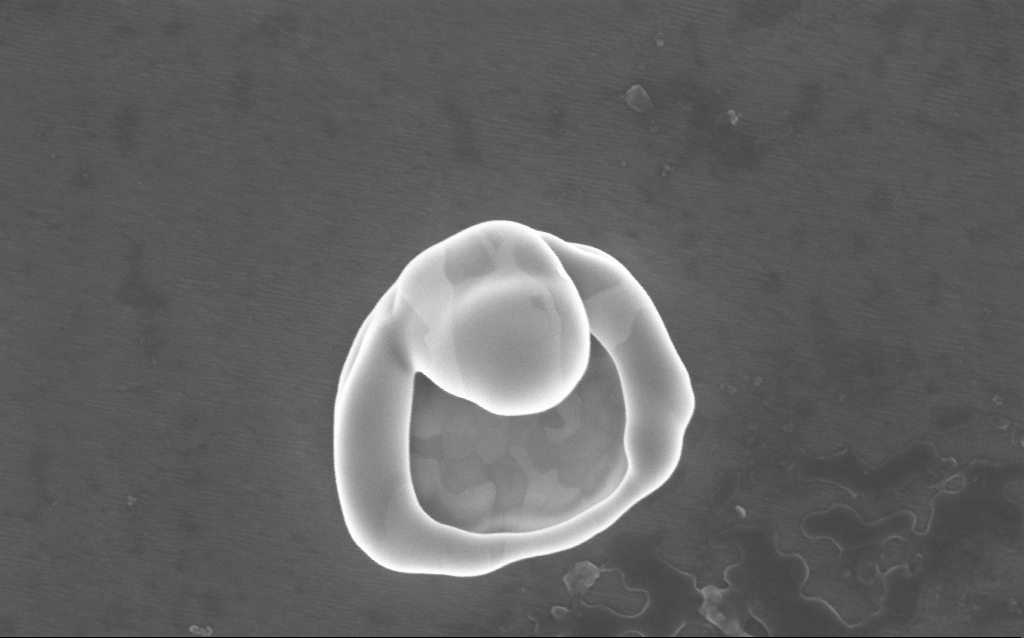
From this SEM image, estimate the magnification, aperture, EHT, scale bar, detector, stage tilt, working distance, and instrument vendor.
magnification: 122.97 K X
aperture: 30 µm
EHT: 5 kV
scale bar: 200 nm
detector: InLens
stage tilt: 0°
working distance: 5 mm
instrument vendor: Zeiss